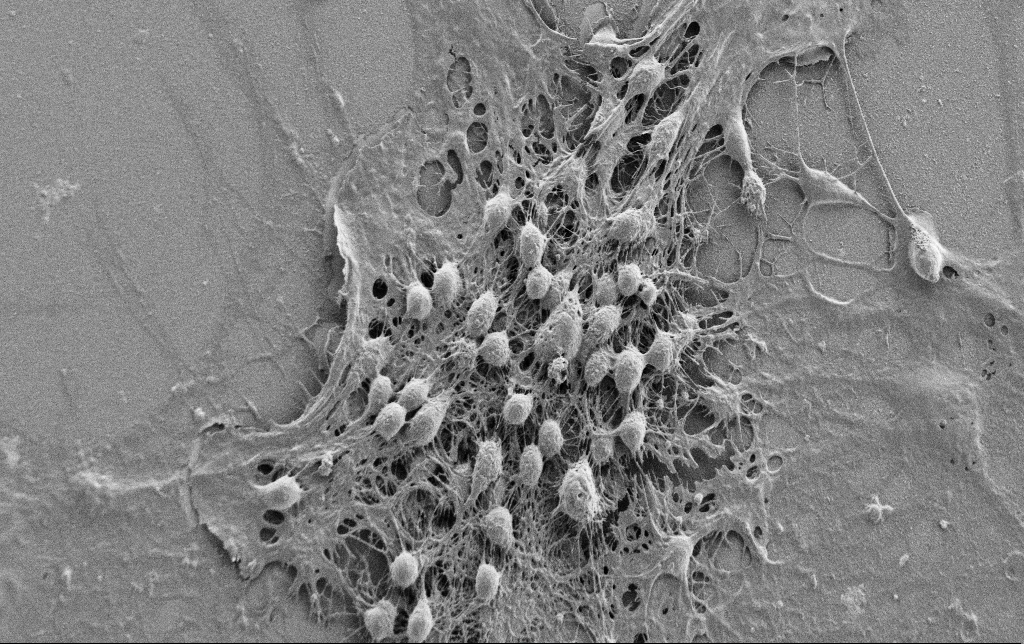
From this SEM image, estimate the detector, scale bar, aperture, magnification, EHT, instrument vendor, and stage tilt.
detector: SE2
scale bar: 10000 nm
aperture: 30 µm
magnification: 2 K X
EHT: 0.9 kV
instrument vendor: Zeiss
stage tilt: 0°